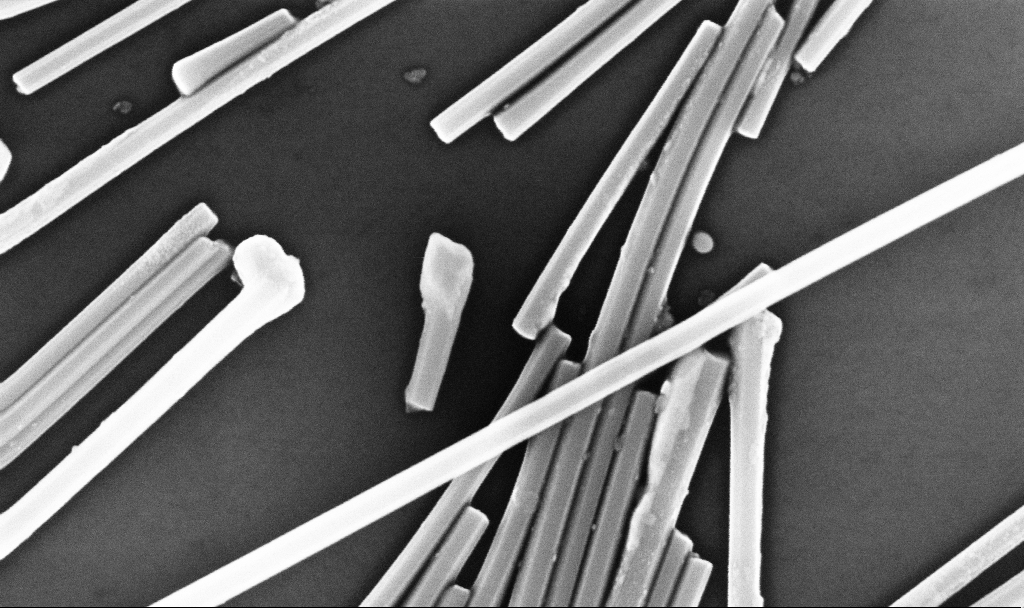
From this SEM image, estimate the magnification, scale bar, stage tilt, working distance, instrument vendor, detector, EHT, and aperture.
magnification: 100 K X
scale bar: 200 nm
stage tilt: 0°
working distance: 6.7 mm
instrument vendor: Zeiss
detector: InLens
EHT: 10 kV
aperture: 30 µm